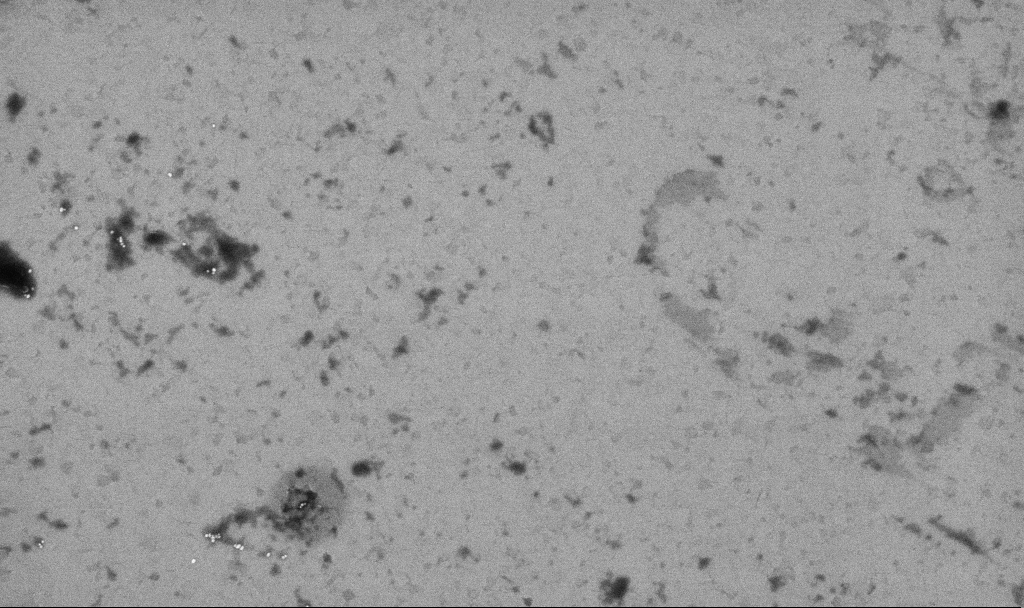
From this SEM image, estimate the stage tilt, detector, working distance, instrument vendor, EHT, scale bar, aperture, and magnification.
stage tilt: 0°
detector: InLens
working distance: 3.3 mm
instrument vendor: Zeiss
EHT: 10 kV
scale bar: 1000 nm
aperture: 30 µm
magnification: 50.38 K X